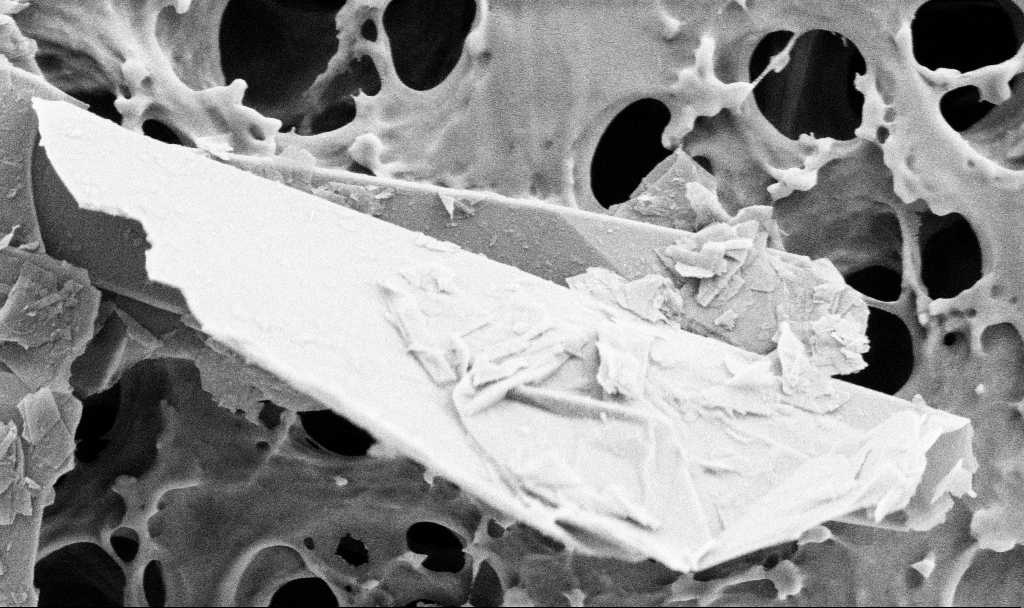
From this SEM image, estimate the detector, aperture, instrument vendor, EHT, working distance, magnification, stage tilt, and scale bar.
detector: SE2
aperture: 30 µm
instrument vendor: Zeiss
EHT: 2 kV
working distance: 3.7 mm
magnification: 50 K X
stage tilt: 0°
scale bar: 1000 nm